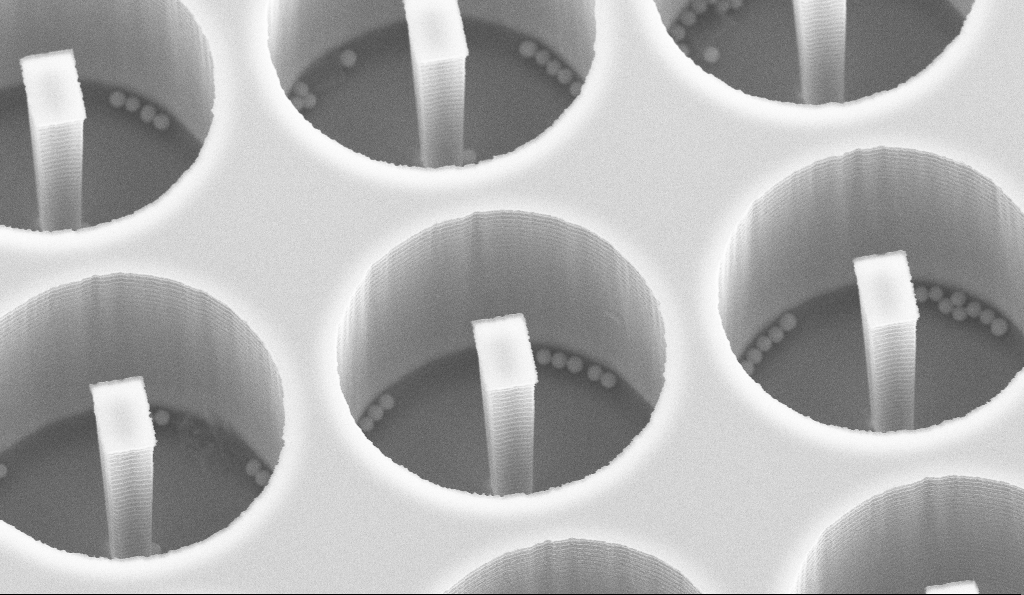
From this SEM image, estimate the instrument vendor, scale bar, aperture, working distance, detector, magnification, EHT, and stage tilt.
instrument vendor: Zeiss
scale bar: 2000 nm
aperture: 30 µm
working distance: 14.1 mm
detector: SE2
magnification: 10 K X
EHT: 10 kV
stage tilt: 30°